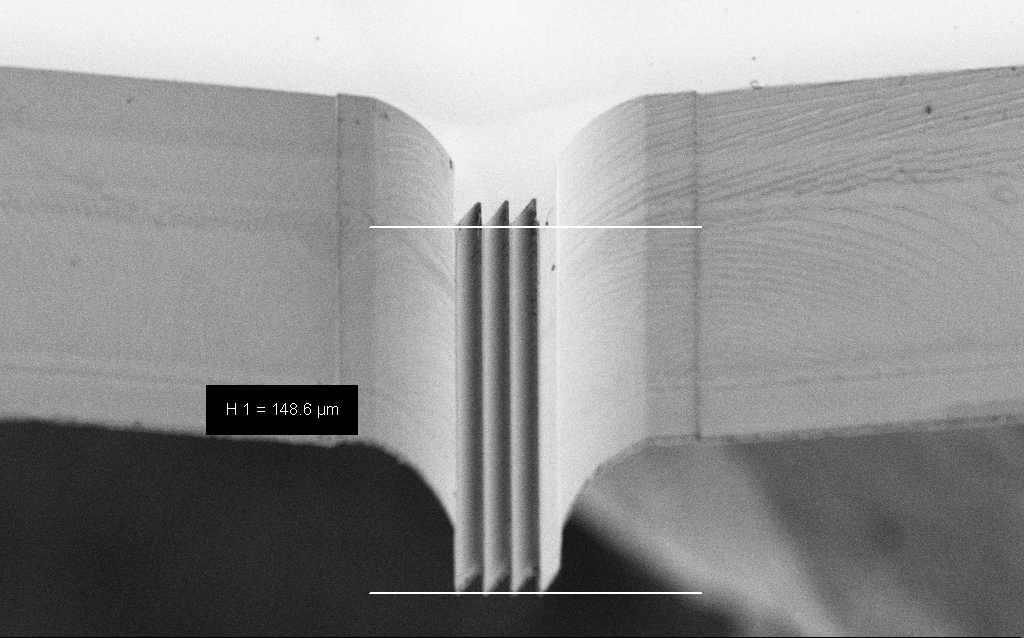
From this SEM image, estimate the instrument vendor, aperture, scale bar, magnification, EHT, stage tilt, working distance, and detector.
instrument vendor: Zeiss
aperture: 30 µm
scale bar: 20000 nm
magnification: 0.904 K X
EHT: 5 kV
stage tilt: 45°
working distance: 6 mm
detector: SE2